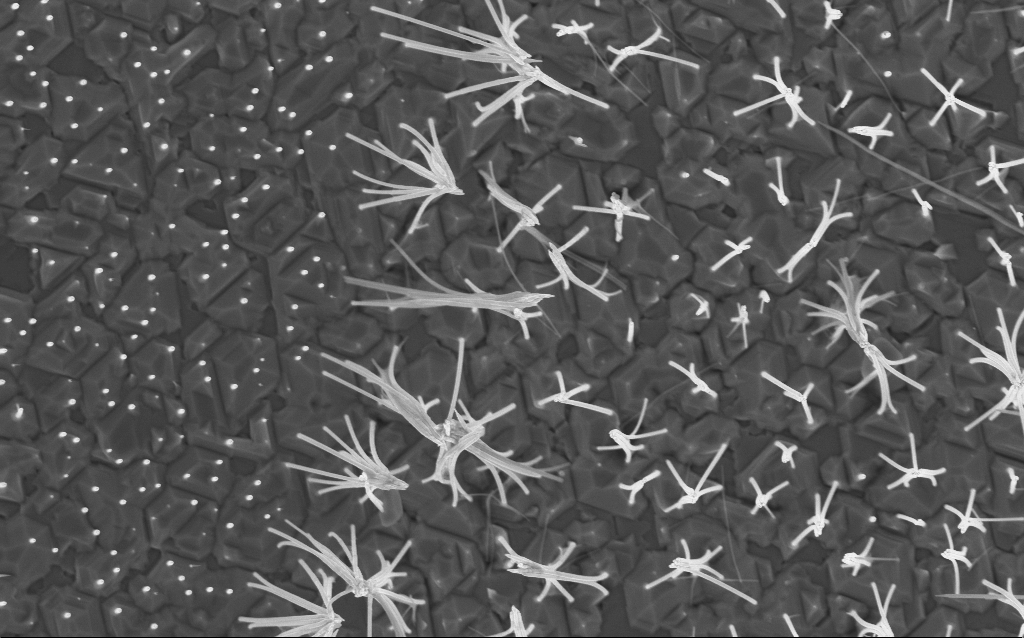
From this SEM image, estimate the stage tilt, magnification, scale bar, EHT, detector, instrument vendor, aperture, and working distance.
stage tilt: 0°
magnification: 20 K X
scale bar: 2000 nm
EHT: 5 kV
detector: InLens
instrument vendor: Zeiss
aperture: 30 µm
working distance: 6.7 mm